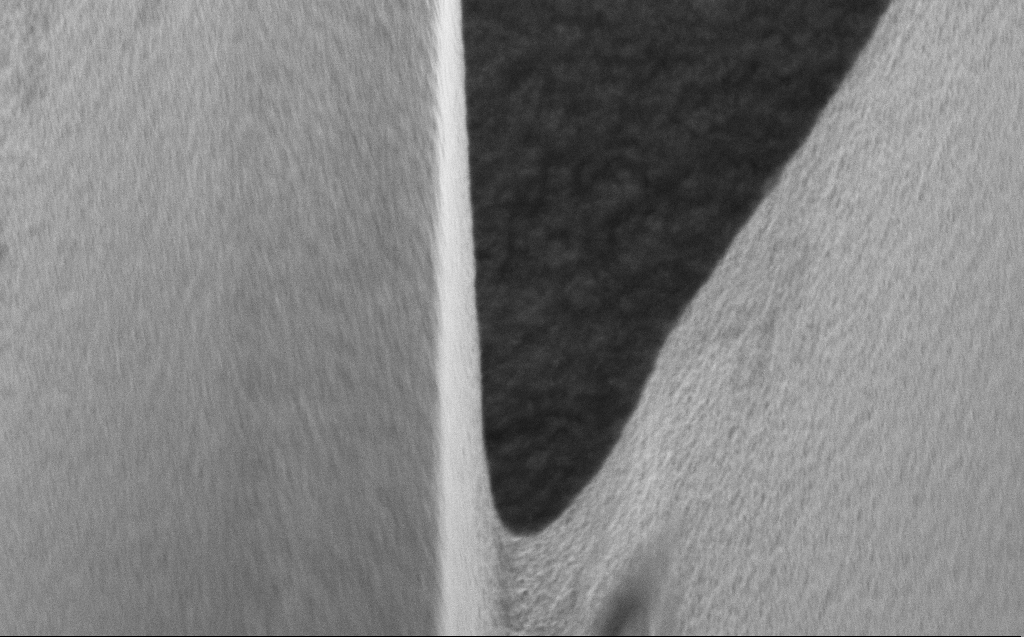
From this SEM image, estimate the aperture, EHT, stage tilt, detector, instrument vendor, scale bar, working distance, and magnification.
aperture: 30 µm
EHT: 3 kV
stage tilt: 45°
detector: InLens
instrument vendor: Zeiss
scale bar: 2000 nm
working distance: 5 mm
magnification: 35.94 K X